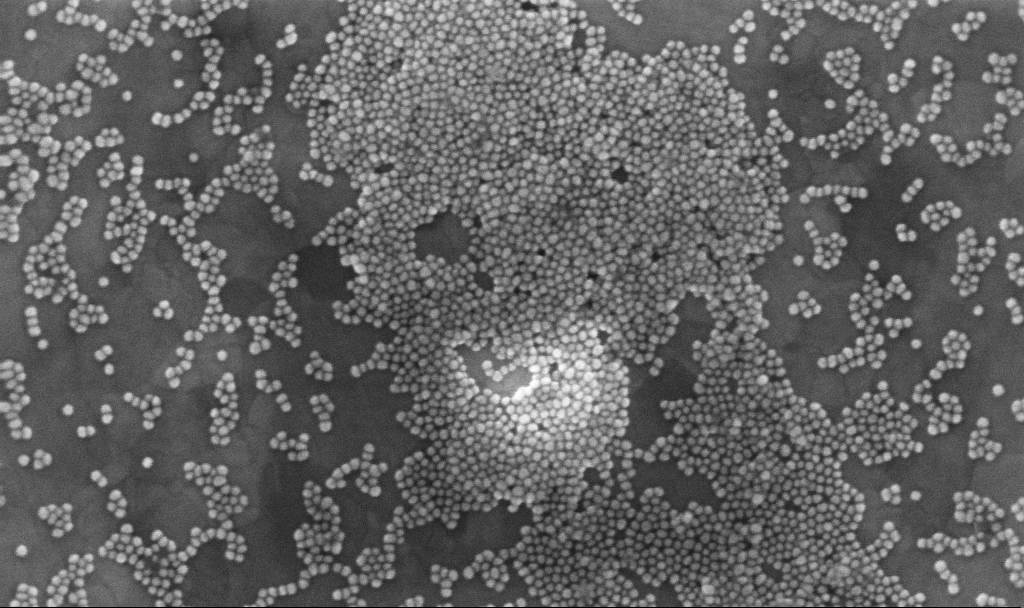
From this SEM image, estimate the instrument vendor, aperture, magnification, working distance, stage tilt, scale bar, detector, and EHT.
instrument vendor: Zeiss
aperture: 30 µm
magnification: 200 K X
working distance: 3.4 mm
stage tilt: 0°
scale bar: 100 nm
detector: InLens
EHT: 10 kV